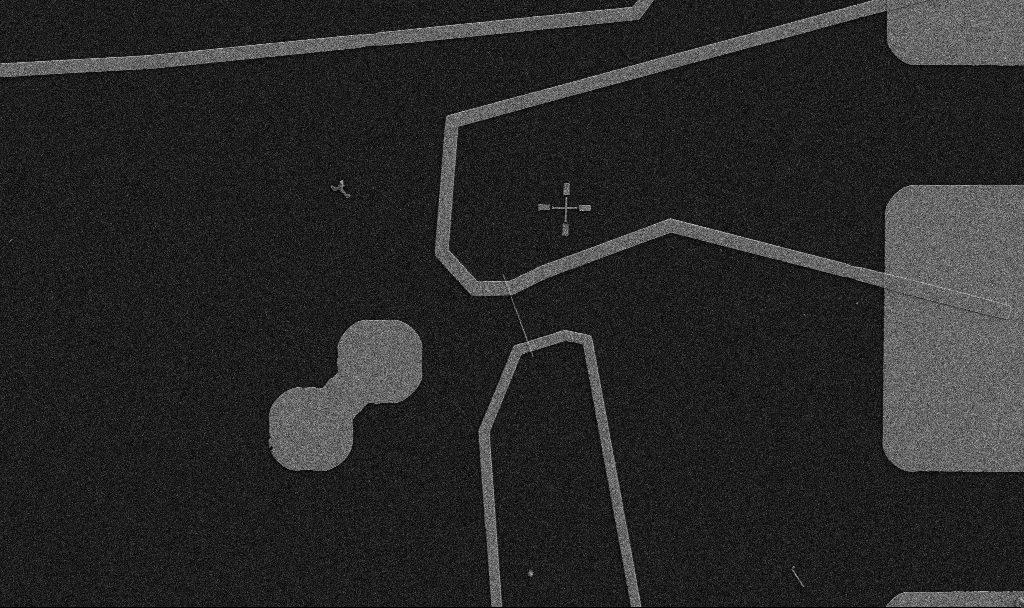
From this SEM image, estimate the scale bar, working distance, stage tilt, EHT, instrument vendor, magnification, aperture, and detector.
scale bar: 10000 nm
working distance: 10.7 mm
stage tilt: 0°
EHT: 5 kV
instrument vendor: Zeiss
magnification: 5 K X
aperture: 30 µm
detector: SE2